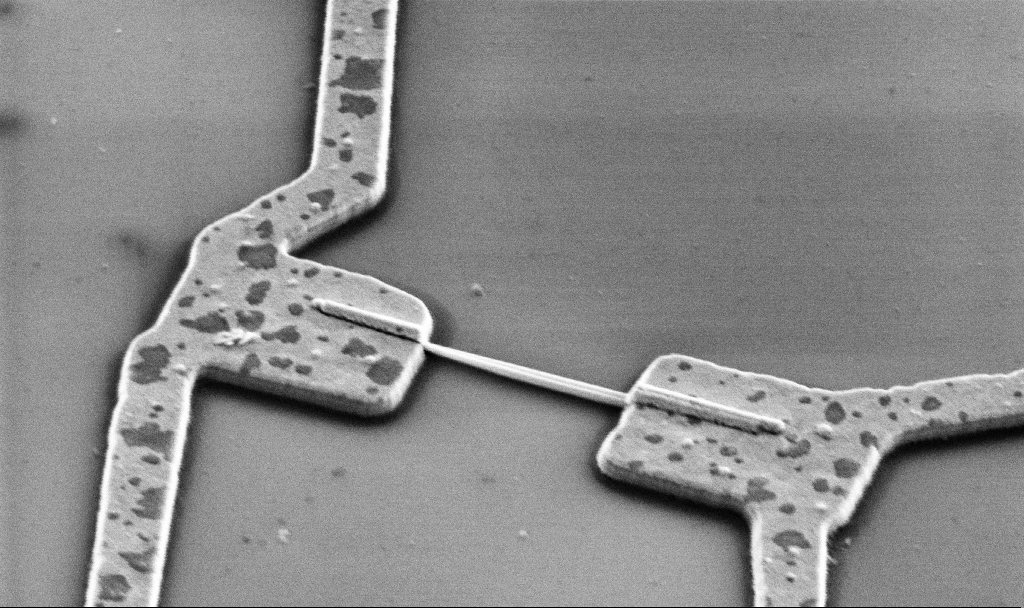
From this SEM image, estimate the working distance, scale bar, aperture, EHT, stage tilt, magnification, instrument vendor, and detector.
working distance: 15.6 mm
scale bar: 1000 nm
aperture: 30 µm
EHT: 5 kV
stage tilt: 45°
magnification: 30 K X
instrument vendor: Zeiss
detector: SE2